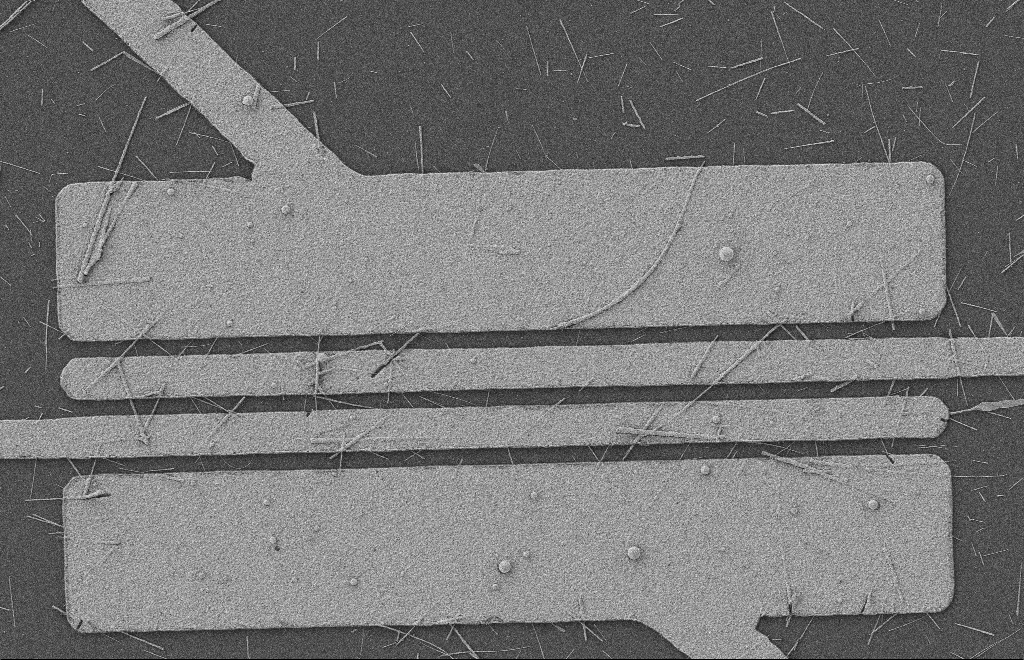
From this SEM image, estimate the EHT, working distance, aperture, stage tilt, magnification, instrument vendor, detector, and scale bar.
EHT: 2 kV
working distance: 8 mm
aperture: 20 µm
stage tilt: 0°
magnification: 5.35 K X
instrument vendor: Zeiss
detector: SE2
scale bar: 2000 nm